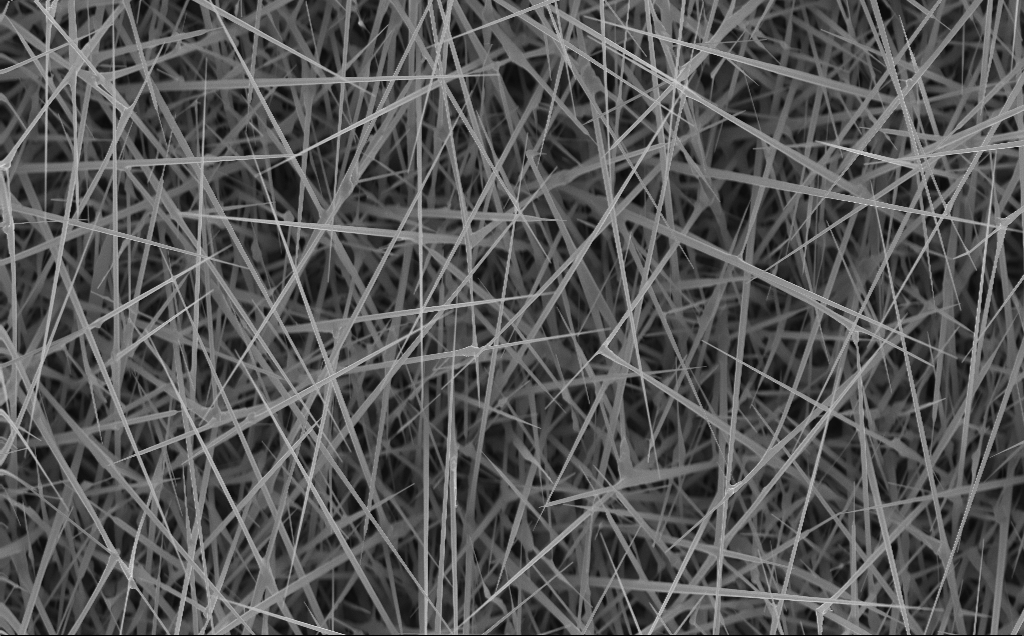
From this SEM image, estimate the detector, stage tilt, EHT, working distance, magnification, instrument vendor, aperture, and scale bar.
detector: InLens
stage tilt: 0°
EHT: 10 kV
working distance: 4 mm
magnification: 10 K X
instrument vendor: Zeiss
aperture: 30 µm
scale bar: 2000 nm